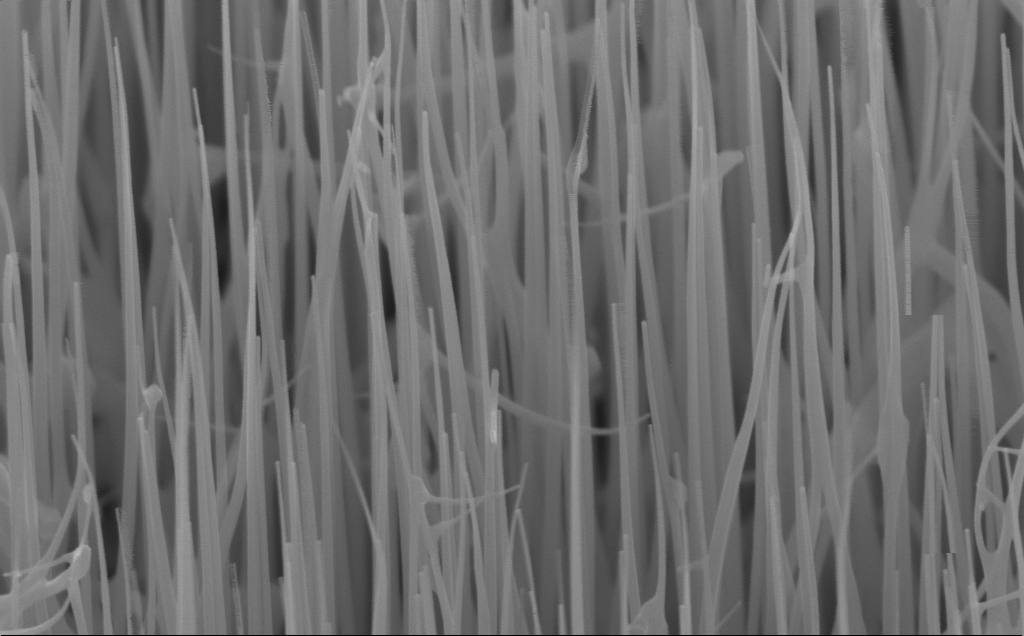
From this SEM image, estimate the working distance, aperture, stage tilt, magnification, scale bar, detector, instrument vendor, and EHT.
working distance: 6 mm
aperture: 30 µm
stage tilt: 45°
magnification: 100 K X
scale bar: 200 nm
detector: InLens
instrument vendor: Zeiss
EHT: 10 kV